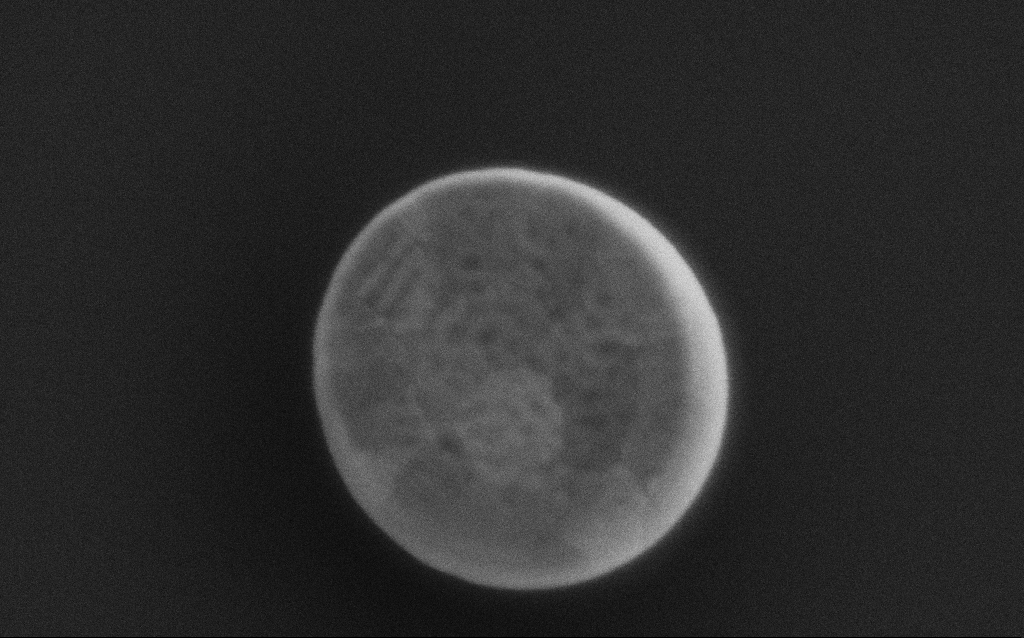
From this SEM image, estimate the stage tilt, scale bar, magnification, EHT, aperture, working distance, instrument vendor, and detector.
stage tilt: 0°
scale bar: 200 nm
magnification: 209.14 K X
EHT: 3 kV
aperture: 30 µm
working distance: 3 mm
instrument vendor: Zeiss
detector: SE2